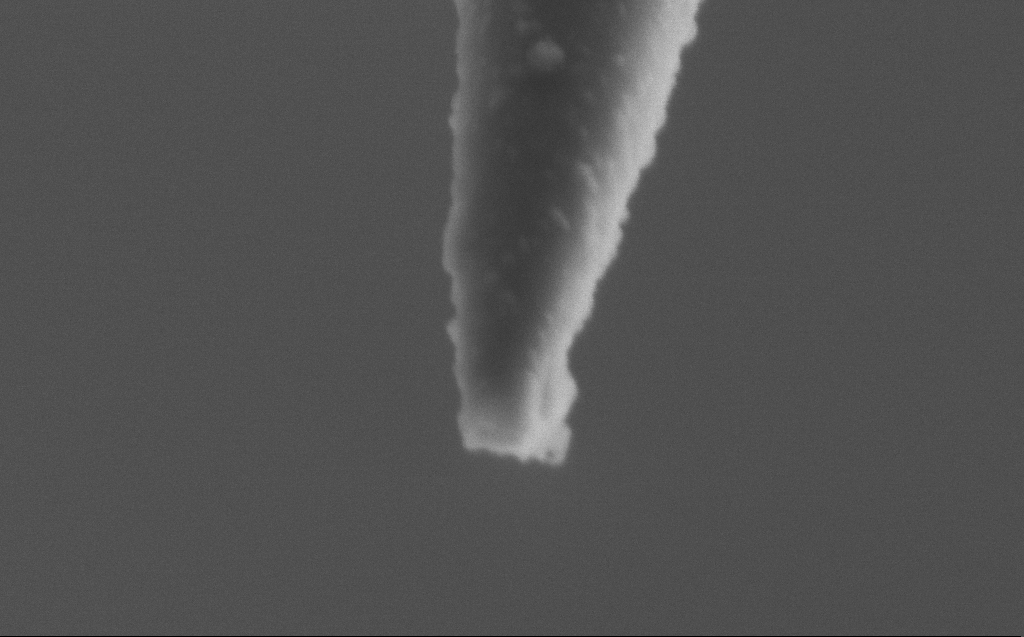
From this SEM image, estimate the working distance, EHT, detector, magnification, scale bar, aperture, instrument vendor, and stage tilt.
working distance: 4 mm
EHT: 2 kV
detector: SE2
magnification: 250 K X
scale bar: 200 nm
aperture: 30 µm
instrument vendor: Zeiss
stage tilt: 45°